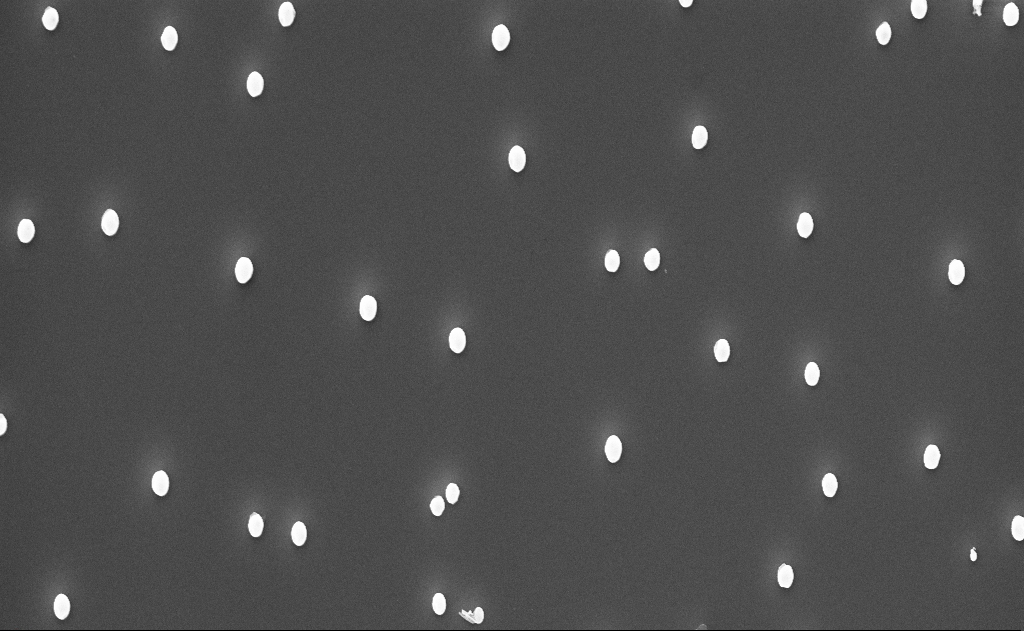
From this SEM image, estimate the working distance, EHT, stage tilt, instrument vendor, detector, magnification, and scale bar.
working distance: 12 mm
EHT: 10 kV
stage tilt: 0°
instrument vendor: Zeiss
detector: InLens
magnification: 20 K X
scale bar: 2000 nm